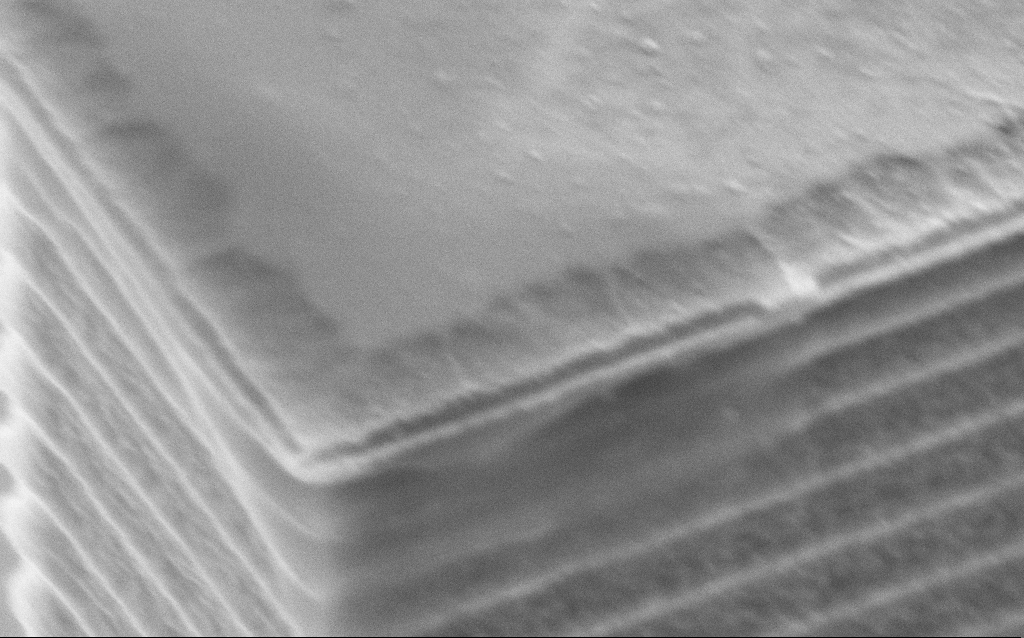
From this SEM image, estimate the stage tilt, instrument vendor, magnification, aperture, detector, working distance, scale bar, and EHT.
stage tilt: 45°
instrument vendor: Zeiss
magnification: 101.07 K X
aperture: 30 µm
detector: SE2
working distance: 12 mm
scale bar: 200 nm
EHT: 5 kV